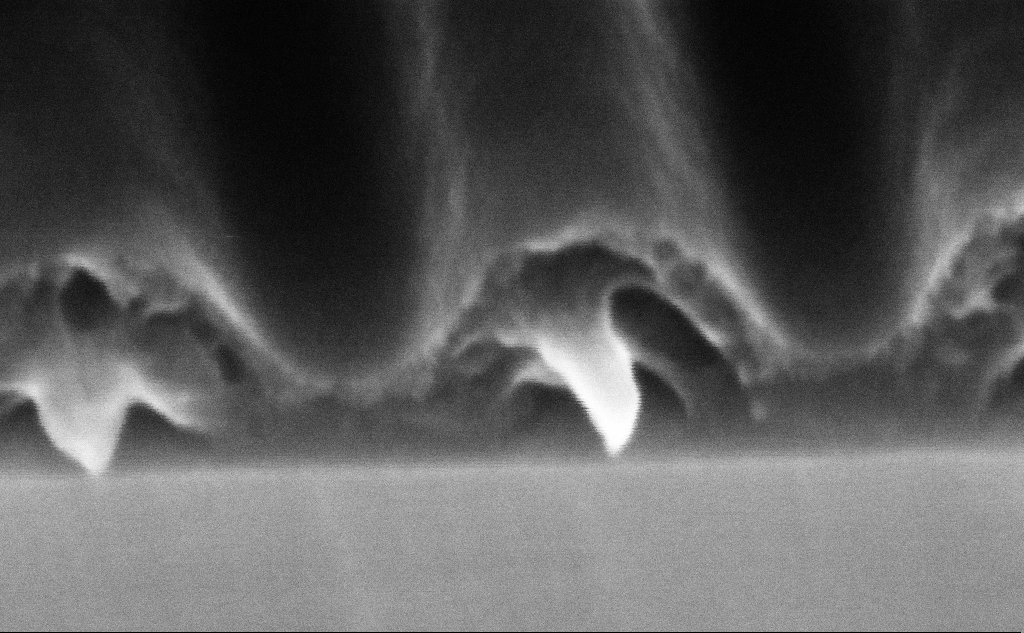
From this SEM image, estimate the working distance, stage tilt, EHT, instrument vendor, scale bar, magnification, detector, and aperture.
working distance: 7.4 mm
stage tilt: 45°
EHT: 5 kV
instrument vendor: Zeiss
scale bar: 100 nm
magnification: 606.2 K X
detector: InLens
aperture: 30 µm